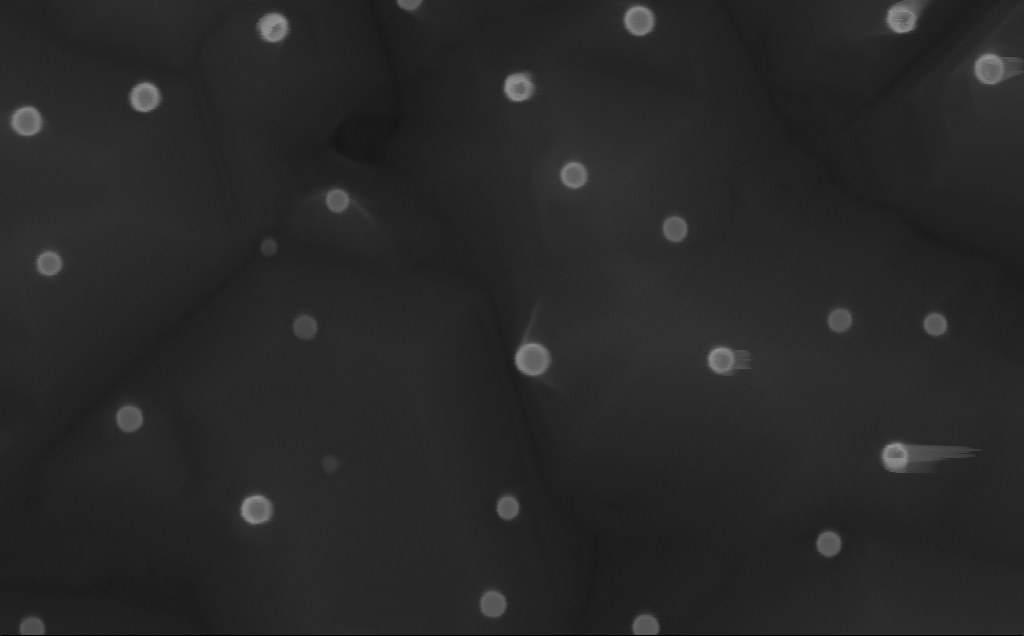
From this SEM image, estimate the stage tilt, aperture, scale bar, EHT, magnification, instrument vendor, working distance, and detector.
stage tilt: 0°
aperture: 30 µm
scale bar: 200 nm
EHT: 10 kV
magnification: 288.78 K X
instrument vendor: Zeiss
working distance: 6 mm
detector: InLens